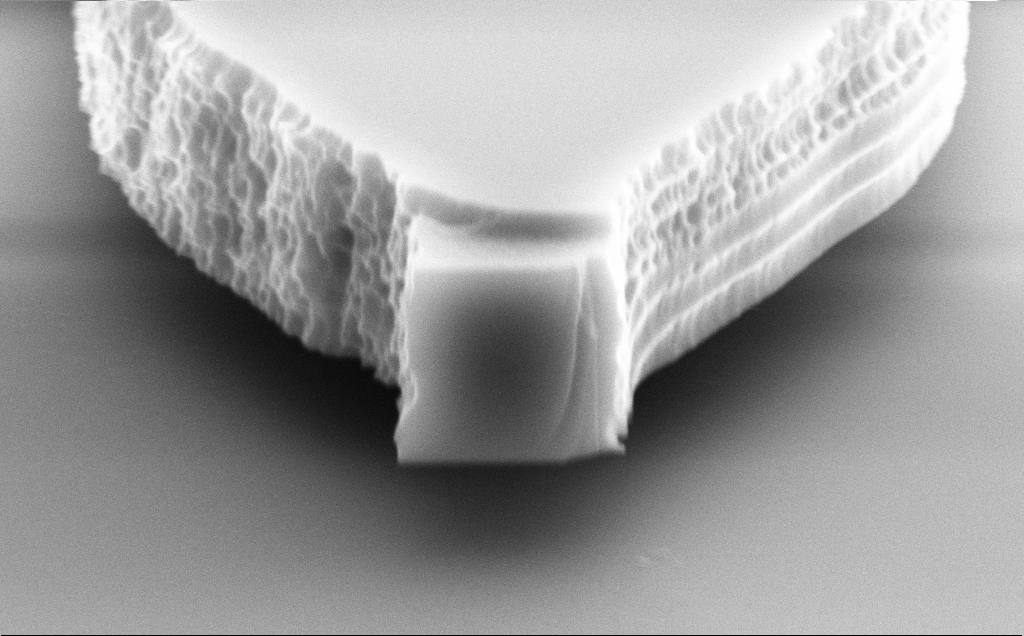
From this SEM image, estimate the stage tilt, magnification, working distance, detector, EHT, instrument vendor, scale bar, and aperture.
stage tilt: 70°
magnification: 34.56 K X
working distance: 9 mm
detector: SE2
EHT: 10 kV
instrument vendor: Zeiss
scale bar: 2000 nm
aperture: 30 µm